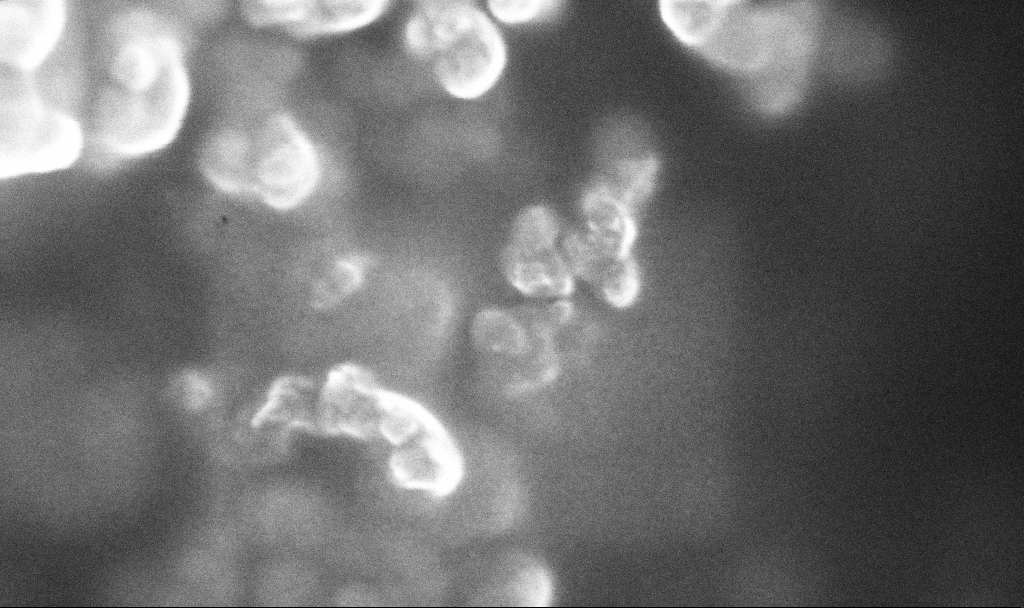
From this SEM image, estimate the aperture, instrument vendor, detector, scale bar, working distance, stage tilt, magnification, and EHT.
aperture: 30 µm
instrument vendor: Zeiss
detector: InLens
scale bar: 200 nm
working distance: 2.4 mm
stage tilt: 0°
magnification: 317.11 K X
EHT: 10 kV